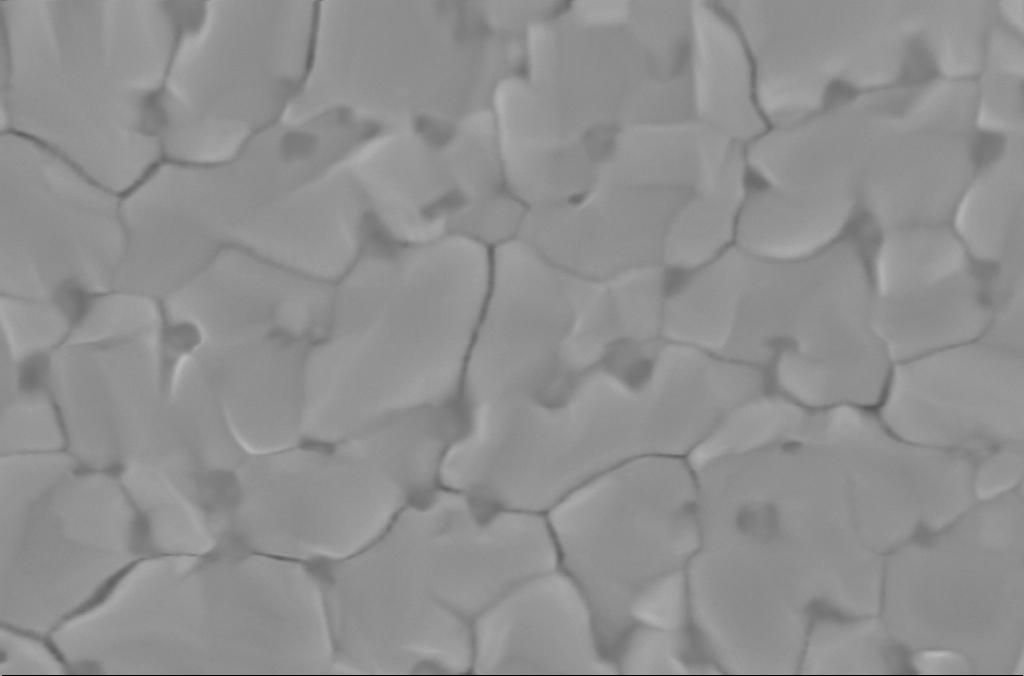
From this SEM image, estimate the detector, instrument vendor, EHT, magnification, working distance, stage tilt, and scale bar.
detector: InLens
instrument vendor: Zeiss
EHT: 5 kV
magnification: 100 K X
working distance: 3 mm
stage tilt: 0°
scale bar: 200 nm